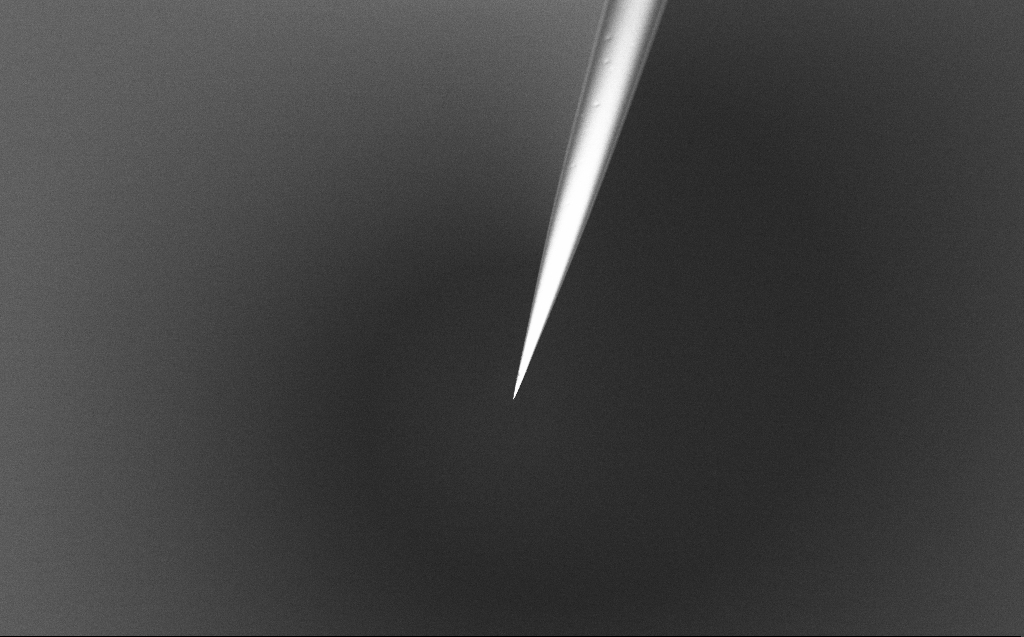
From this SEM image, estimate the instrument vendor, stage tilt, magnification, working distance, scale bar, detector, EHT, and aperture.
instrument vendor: Zeiss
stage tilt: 45°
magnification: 1 K X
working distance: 5 mm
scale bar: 20000 nm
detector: InLens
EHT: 1 kV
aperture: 30 µm